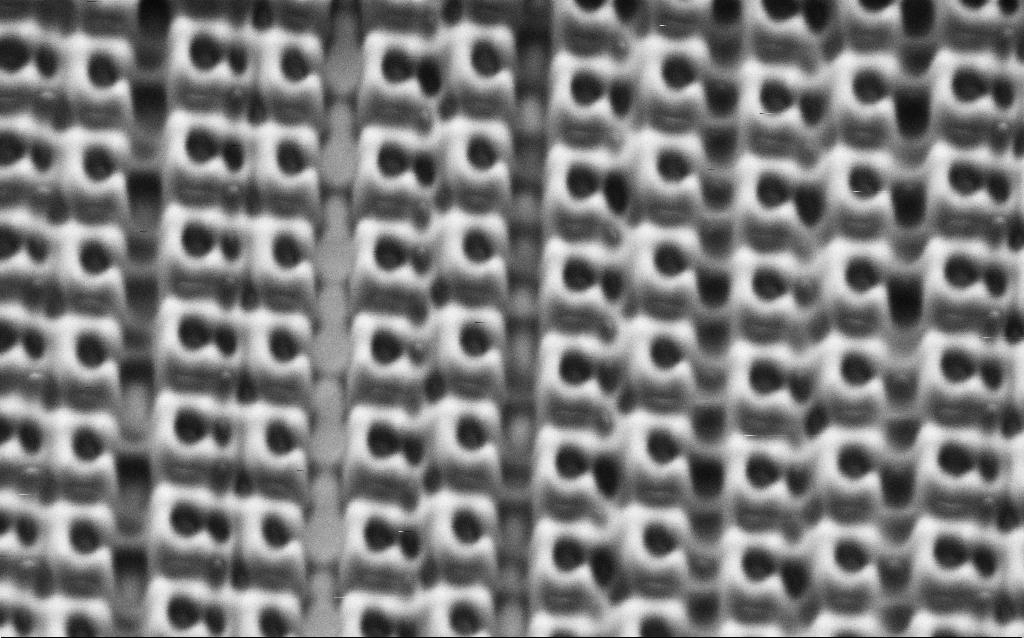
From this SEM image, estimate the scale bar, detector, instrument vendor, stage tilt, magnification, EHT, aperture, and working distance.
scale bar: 1000 nm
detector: SE2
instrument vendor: Zeiss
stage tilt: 30°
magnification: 71.68 K X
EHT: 1.5 kV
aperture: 30 µm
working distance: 7.2 mm